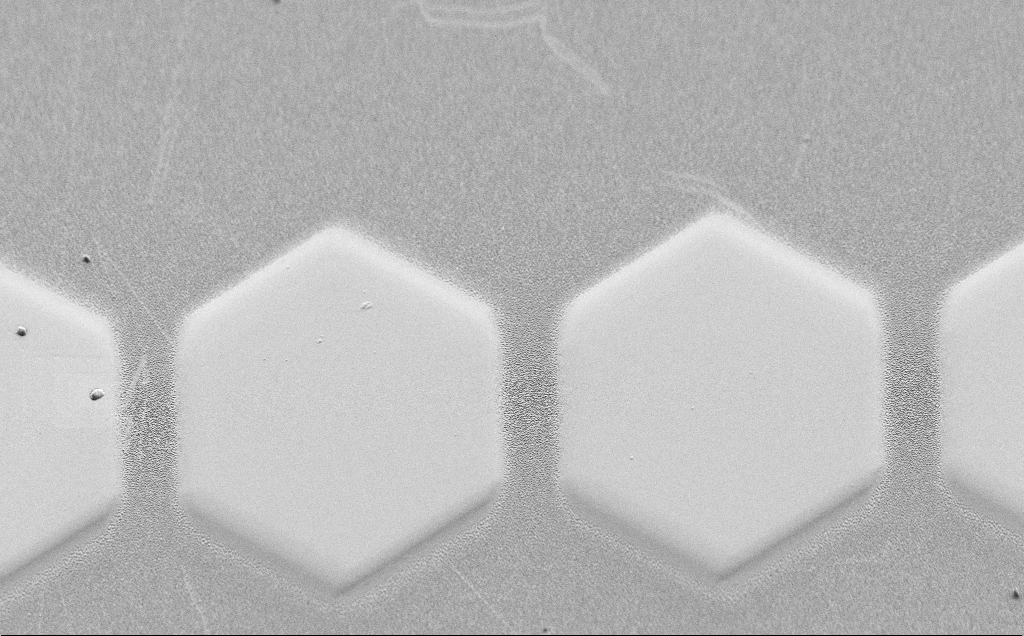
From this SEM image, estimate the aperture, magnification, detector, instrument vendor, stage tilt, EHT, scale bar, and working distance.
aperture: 30 µm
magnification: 2.39 K X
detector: SE2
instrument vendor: Zeiss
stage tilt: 45°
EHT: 1.5 kV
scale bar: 10000 nm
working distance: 4 mm